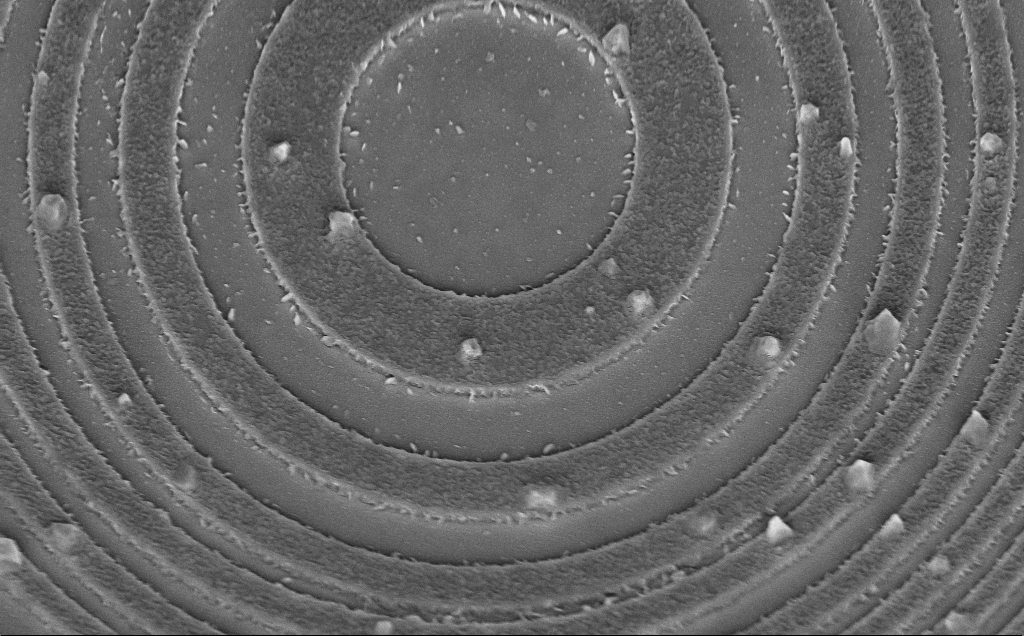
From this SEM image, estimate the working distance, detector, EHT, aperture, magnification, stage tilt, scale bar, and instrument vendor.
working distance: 6 mm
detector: InLens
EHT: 5 kV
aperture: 30 µm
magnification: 14.84 K X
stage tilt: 45°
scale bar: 2000 nm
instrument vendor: Zeiss